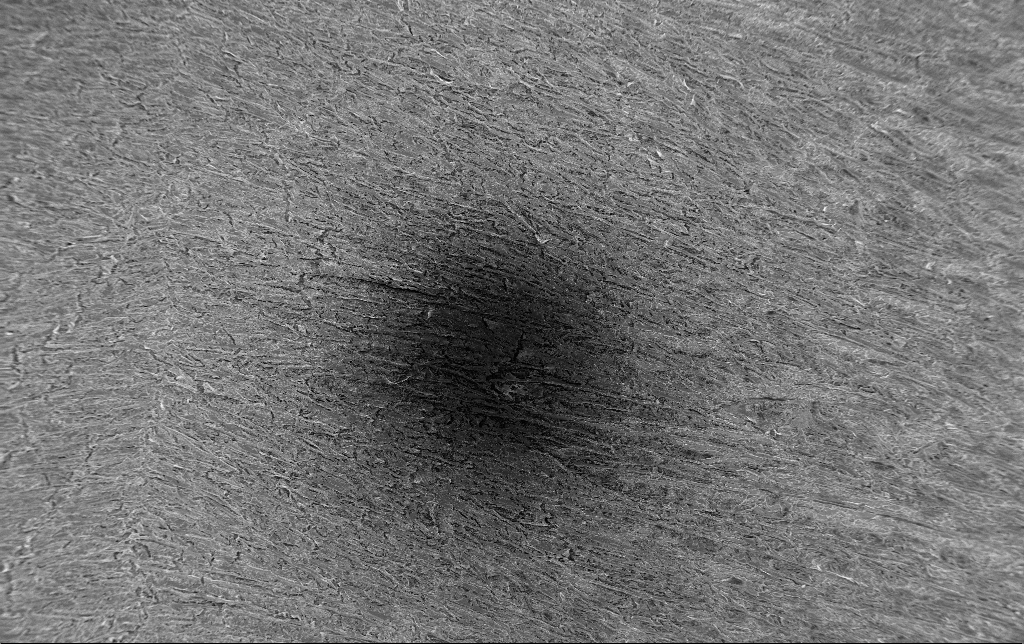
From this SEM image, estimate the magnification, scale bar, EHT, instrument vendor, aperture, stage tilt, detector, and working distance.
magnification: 0.197 K X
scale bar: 100000 nm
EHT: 1.5 kV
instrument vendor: Zeiss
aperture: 30 µm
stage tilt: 0°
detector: InLens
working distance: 3.2 mm